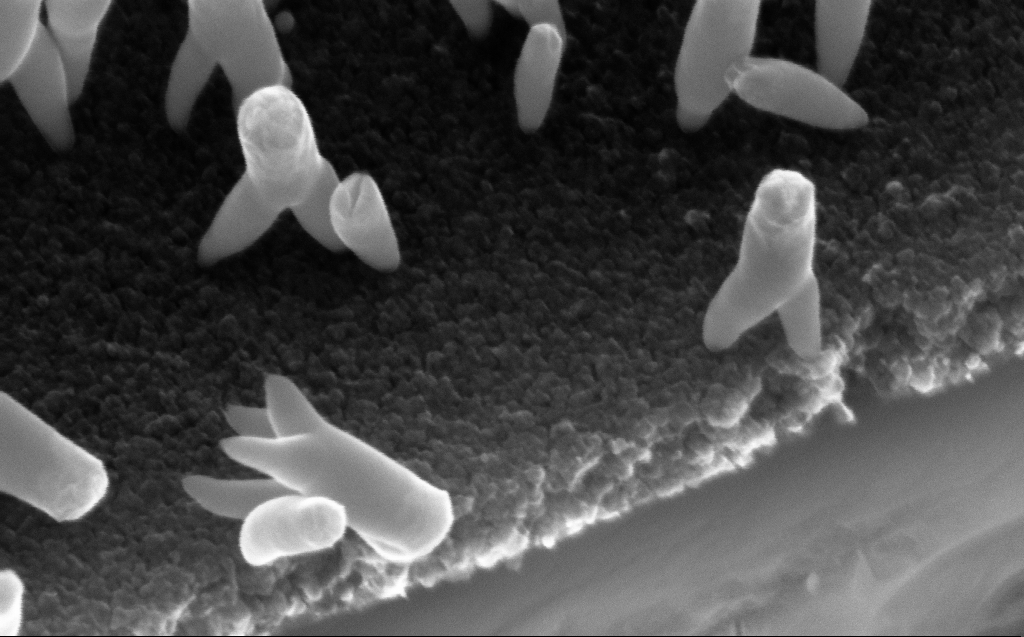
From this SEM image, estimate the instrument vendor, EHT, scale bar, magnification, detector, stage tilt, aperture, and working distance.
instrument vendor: Zeiss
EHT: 10 kV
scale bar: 200 nm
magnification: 215.1 K X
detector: InLens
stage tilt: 45°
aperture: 30 µm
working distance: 5 mm